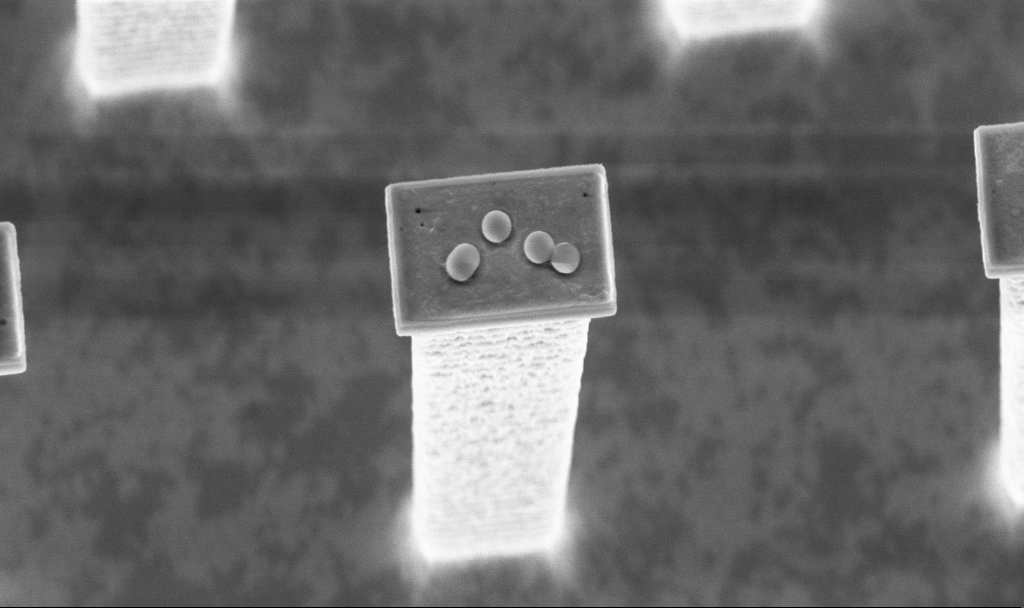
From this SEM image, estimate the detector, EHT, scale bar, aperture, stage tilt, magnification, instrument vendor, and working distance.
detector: InLens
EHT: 5 kV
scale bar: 2000 nm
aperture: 30 µm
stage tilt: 20°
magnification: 18.04 K X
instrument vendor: Zeiss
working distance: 3.2 mm